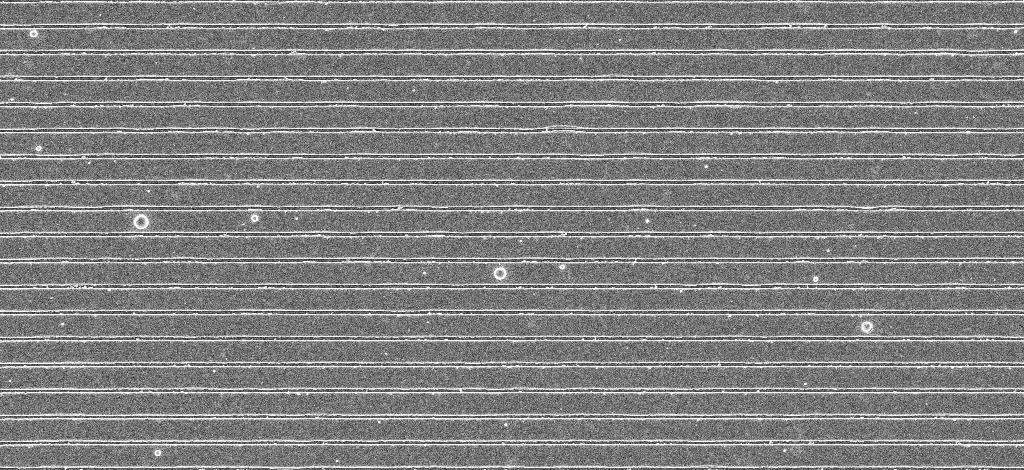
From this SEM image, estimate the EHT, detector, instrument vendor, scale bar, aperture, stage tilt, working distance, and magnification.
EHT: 5 kV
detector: InLens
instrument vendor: Zeiss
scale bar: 2000 nm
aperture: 30 µm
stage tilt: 0°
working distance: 5.2 mm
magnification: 12.04 K X